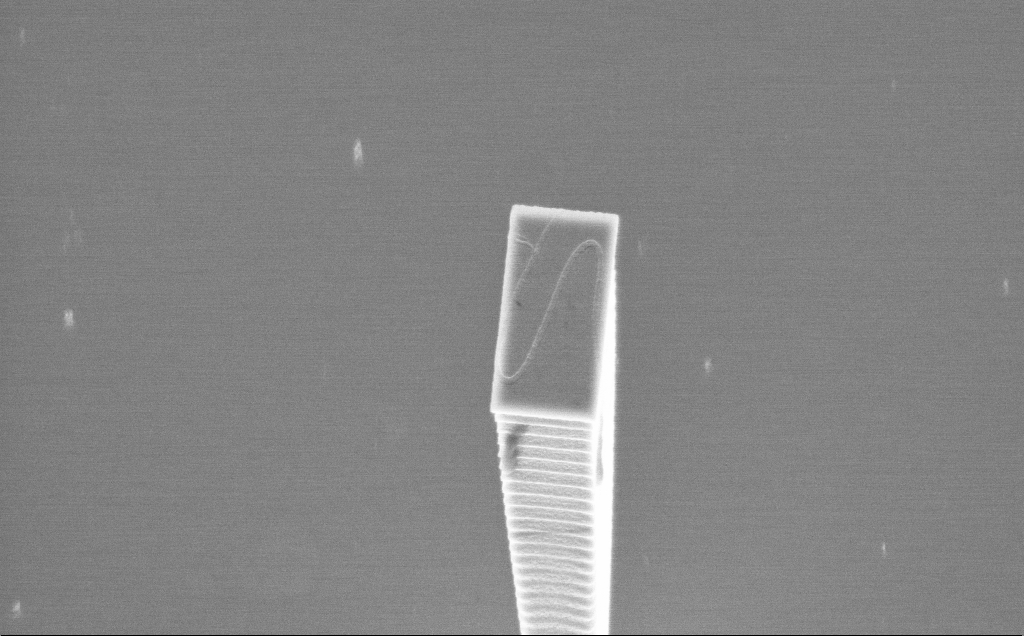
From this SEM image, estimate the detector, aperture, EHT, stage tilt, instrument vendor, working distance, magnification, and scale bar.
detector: InLens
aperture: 30 µm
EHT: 5 kV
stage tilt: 36.8°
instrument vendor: Zeiss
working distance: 3 mm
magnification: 13.92 K X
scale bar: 2000 nm